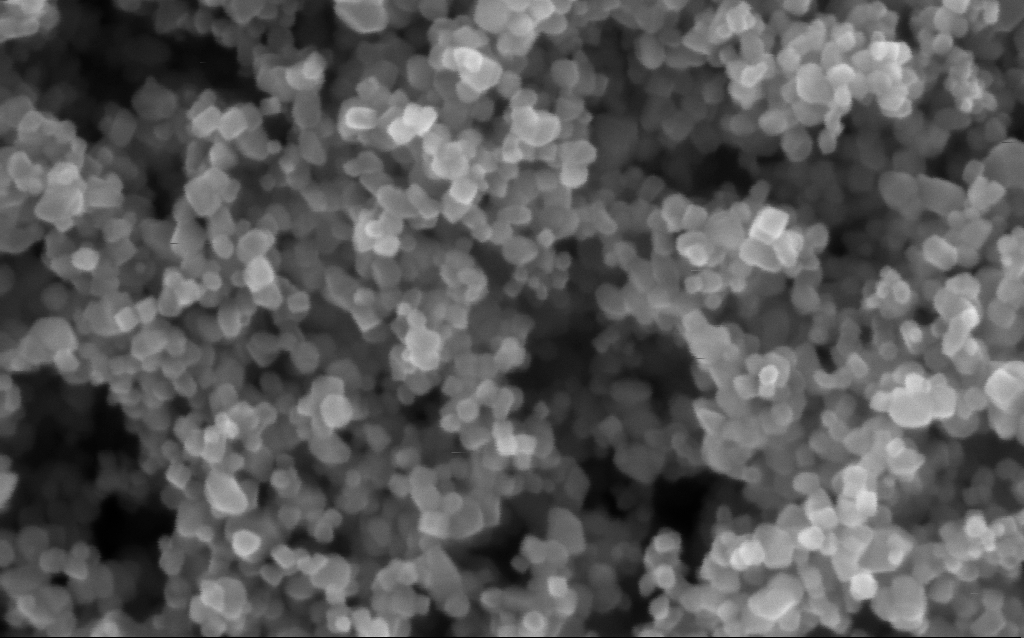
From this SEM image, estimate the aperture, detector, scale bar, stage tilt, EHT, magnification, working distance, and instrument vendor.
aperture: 30 µm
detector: InLens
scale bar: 100 nm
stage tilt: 0°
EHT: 5 kV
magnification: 416 K X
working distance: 2.9 mm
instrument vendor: Zeiss